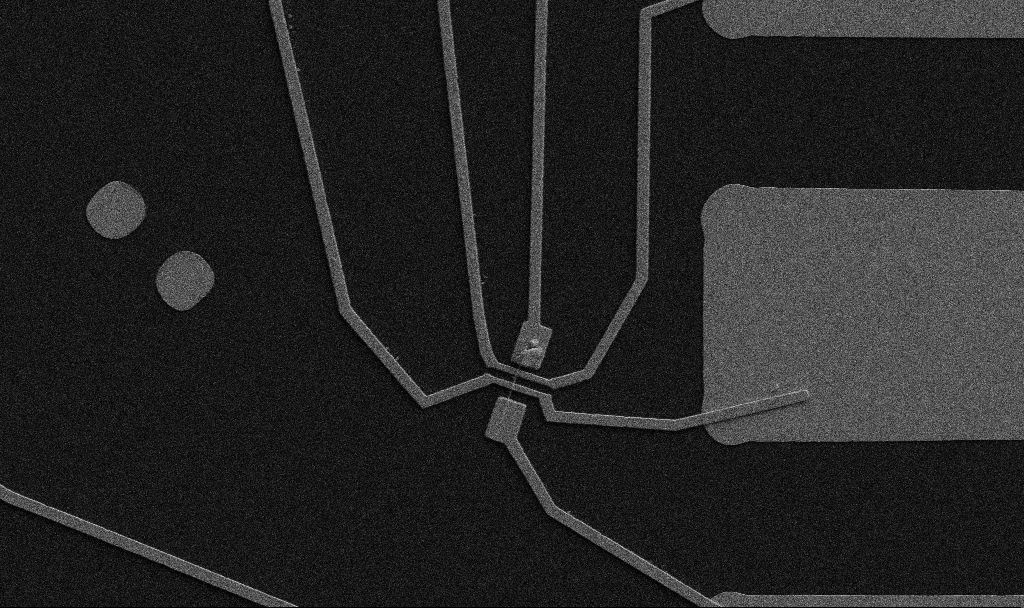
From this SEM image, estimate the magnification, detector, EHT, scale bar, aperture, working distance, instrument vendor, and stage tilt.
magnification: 5 K X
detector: SE2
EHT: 5 kV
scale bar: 10000 nm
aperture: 30 µm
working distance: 10.7 mm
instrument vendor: Zeiss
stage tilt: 0°